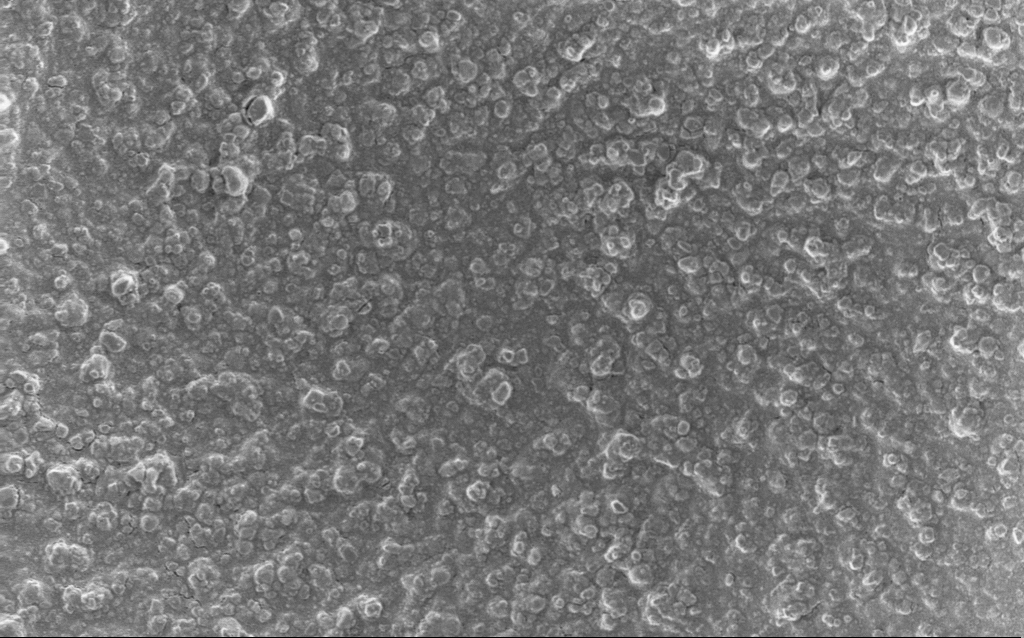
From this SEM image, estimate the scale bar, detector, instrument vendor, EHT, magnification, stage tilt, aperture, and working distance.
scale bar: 20000 nm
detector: InLens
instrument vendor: Zeiss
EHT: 5 kV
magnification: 0.77 K X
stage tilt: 0°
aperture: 30 µm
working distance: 2.9 mm